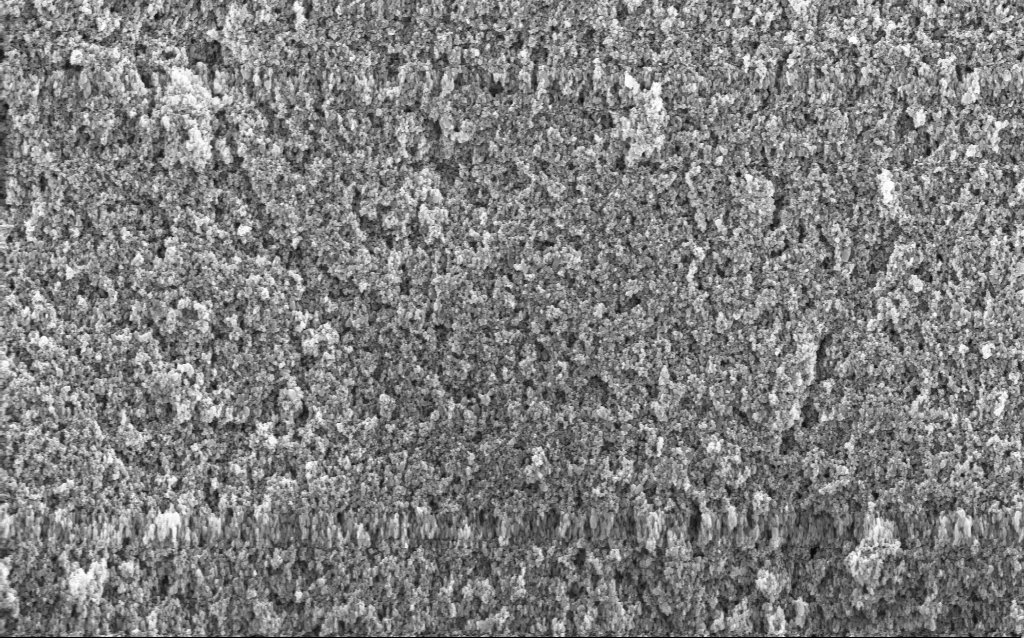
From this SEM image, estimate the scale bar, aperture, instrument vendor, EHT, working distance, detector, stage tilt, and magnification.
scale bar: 1000 nm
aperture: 30 µm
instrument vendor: Zeiss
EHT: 5 kV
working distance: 4.6 mm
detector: InLens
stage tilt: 0°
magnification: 27.75 K X